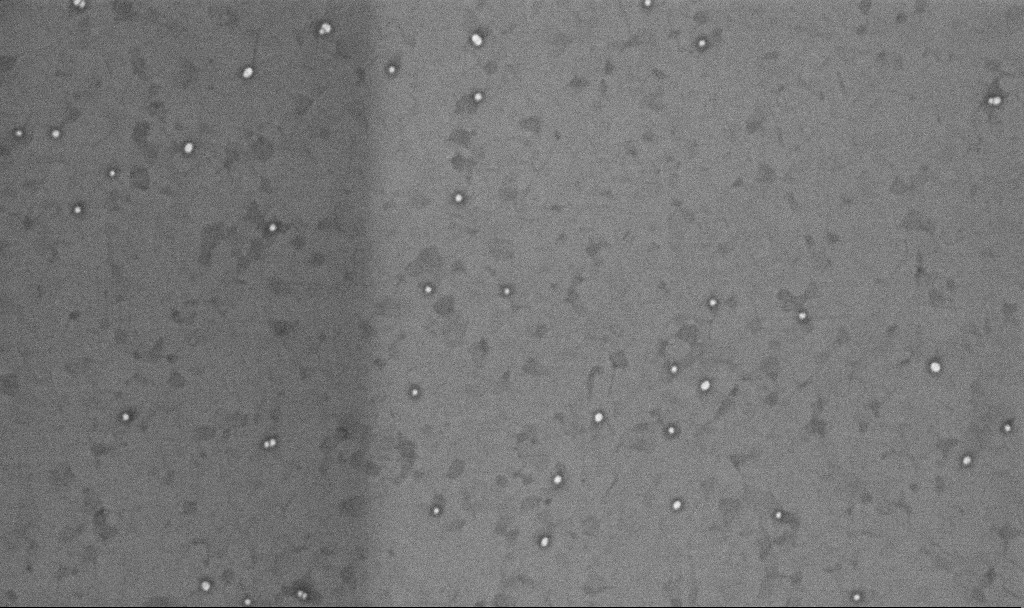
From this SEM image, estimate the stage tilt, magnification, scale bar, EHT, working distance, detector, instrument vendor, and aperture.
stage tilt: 0°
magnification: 100.38 K X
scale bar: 200 nm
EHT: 10 kV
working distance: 3.3 mm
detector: InLens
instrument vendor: Zeiss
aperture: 30 µm